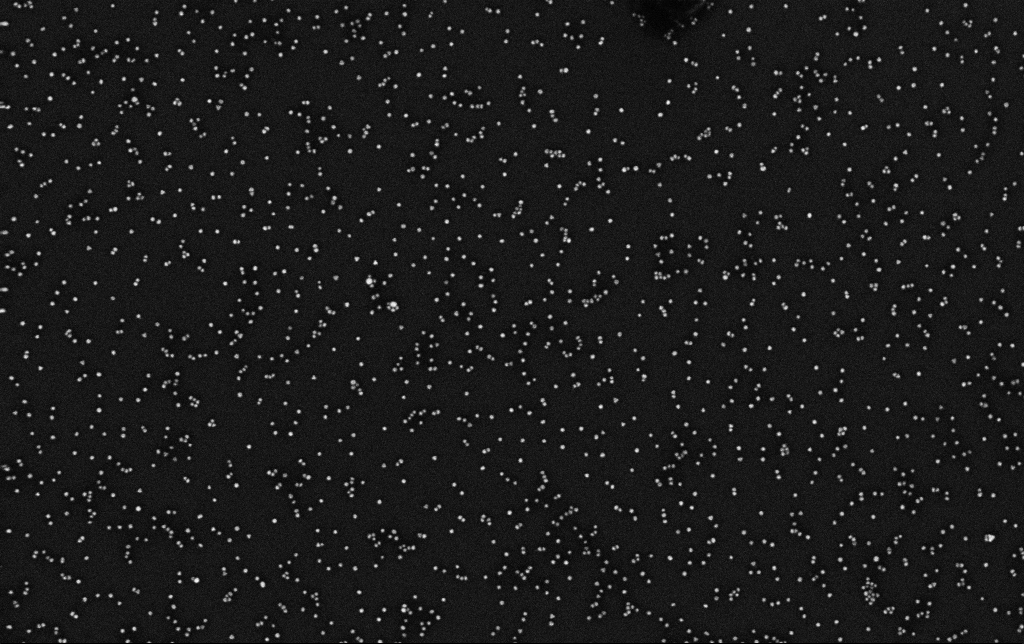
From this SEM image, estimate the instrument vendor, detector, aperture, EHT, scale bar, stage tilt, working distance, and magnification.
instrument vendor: Zeiss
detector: InLens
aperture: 30 µm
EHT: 10 kV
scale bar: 200 nm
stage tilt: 0°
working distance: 3.3 mm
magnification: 100 K X